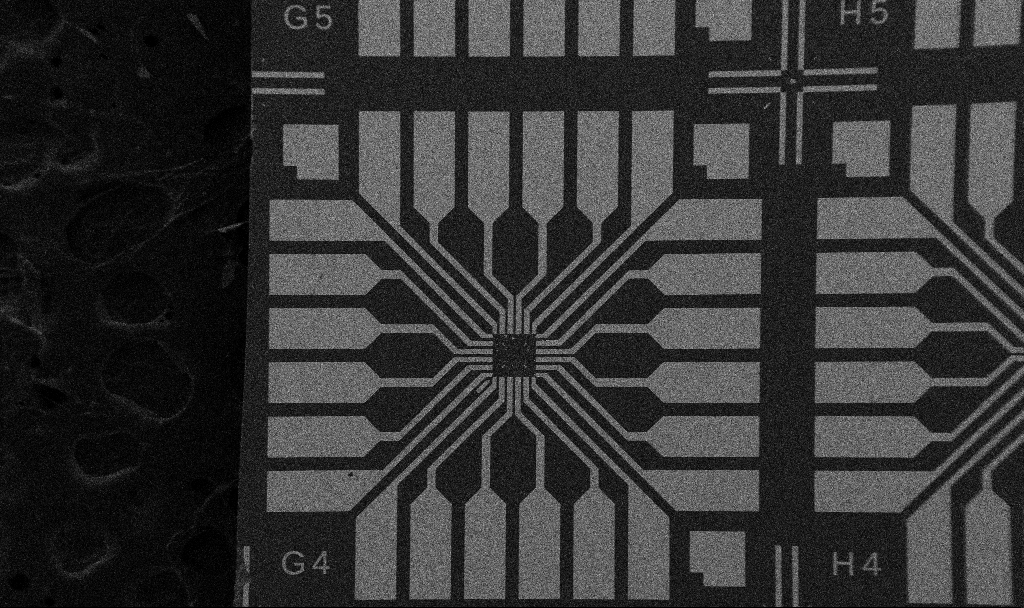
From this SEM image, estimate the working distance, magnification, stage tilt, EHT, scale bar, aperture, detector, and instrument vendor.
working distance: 10.7 mm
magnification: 0.1 K X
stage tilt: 0°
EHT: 5 kV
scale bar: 200000 nm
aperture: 30 µm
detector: SE2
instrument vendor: Zeiss